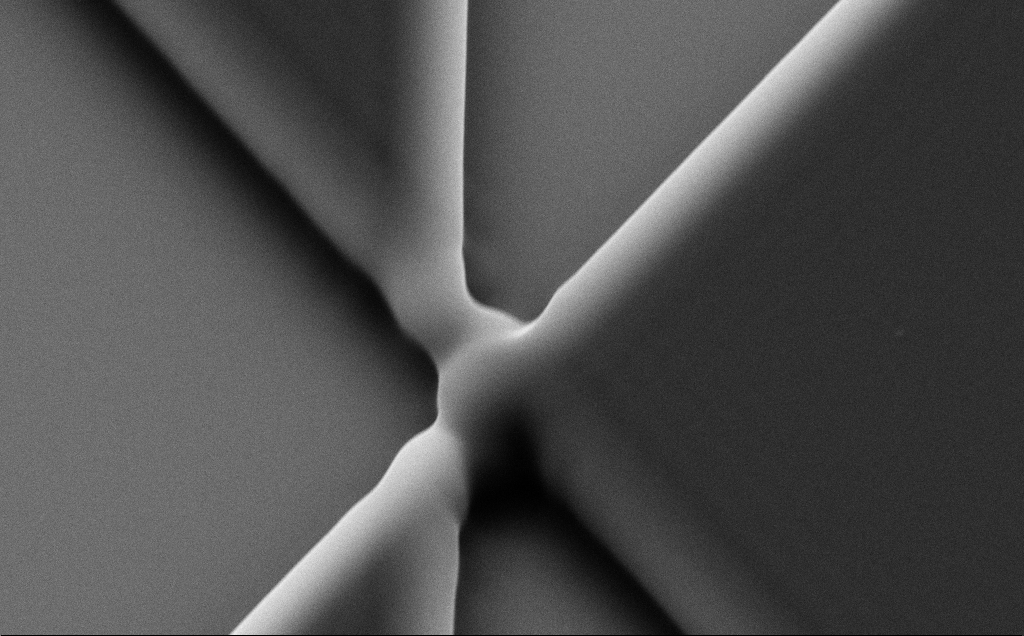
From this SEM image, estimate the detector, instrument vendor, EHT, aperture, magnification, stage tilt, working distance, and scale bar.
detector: SE2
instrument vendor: Zeiss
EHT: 10 kV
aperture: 30 µm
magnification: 17.38 K X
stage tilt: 35°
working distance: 8 mm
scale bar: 2000 nm